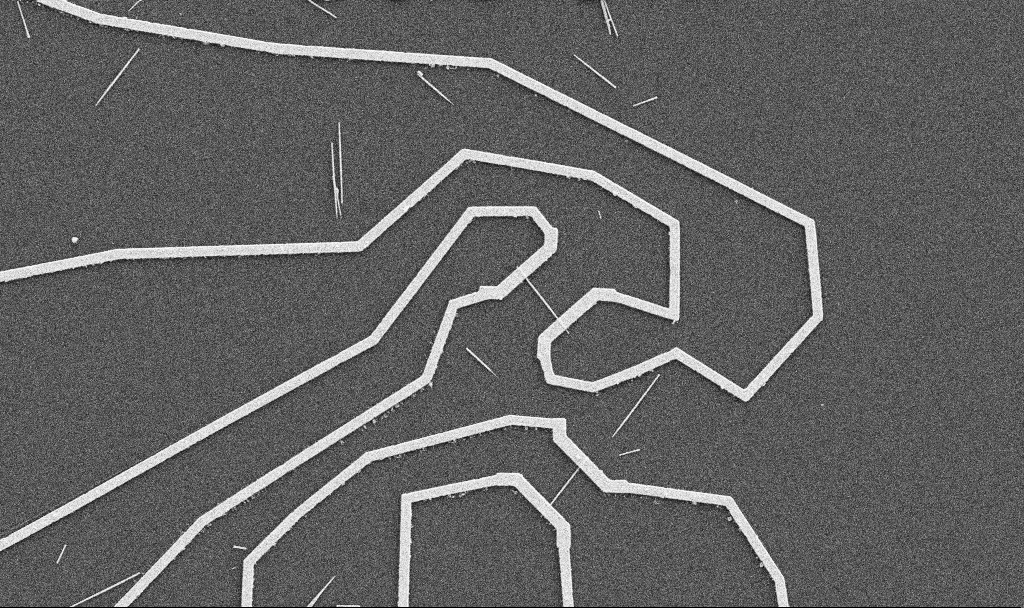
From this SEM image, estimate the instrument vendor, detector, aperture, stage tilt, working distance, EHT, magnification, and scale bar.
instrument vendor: Zeiss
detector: SE2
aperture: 30 µm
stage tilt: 0°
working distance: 10.7 mm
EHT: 5 kV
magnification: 5 K X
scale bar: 10000 nm